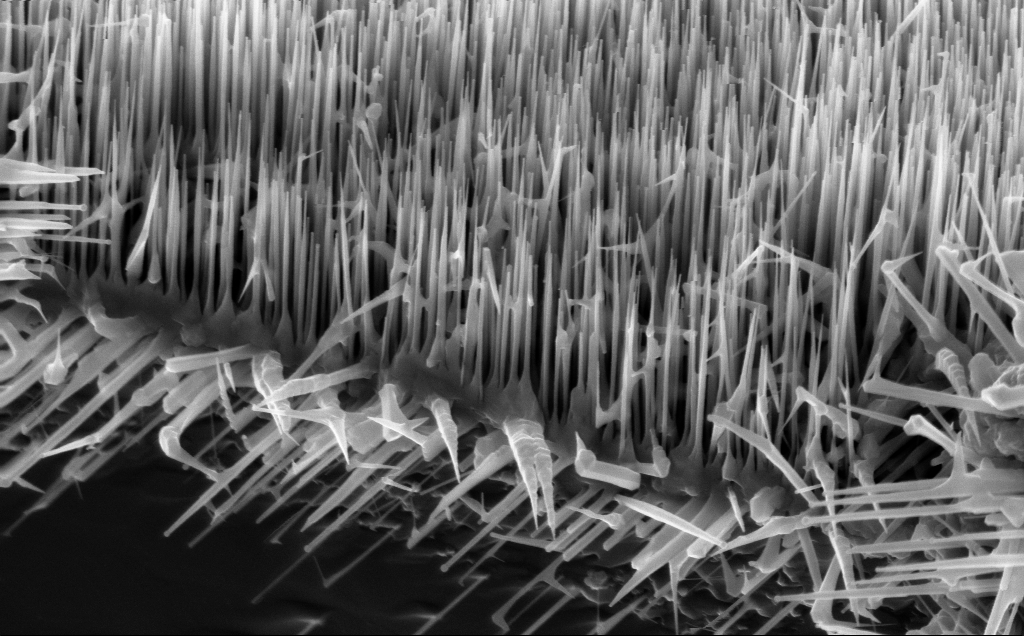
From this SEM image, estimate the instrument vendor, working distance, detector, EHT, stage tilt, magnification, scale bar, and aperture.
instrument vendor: Zeiss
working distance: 5 mm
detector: InLens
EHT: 10 kV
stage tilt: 45°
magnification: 47.08 K X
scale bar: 1000 nm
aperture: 30 µm